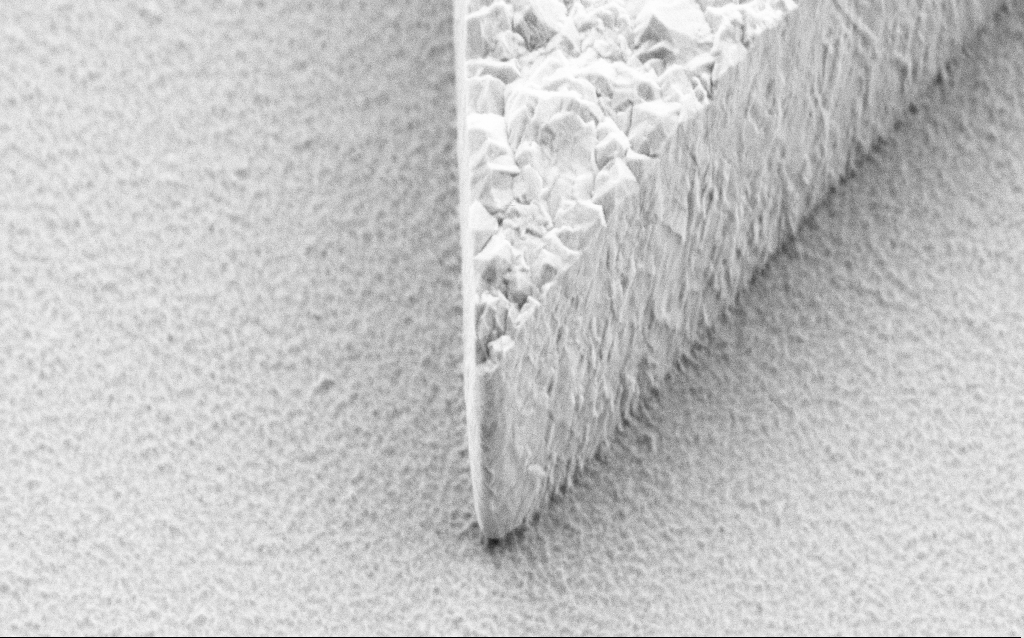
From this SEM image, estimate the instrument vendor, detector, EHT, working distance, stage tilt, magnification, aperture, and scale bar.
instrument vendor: Zeiss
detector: SE2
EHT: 3 kV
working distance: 6.4 mm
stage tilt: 45°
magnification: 61.01 K X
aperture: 30 µm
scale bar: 1000 nm